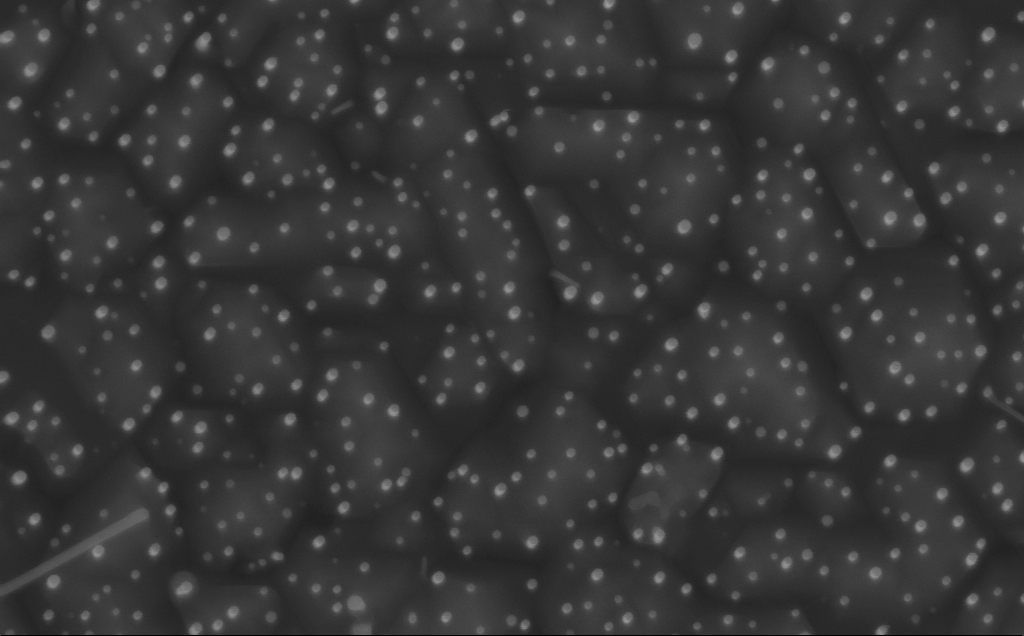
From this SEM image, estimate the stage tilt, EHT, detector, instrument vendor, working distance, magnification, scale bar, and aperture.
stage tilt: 0°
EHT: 10 kV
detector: InLens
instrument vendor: Zeiss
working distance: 5 mm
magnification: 80 K X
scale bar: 200 nm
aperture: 30 µm